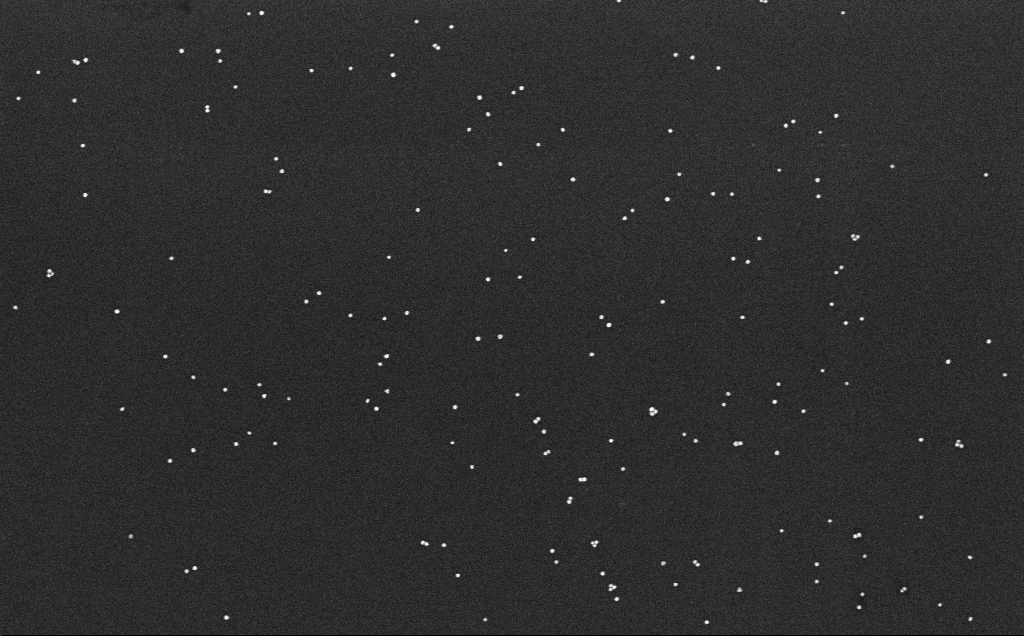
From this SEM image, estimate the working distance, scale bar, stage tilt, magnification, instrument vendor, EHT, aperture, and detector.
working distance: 6.6 mm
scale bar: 200 nm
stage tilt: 0°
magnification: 100 K X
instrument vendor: Zeiss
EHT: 10 kV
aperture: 30 µm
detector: InLens